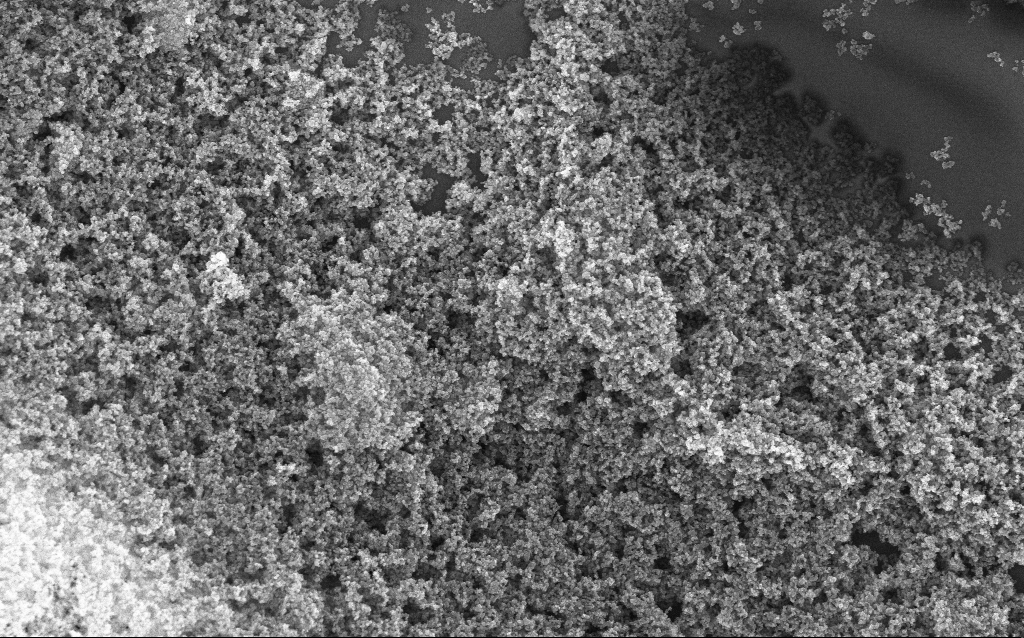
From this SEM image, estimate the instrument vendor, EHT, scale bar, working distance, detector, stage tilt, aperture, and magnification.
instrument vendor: Zeiss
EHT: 10 kV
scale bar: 1000 nm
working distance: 2.4 mm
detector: InLens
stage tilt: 0°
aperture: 30 µm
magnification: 37.88 K X